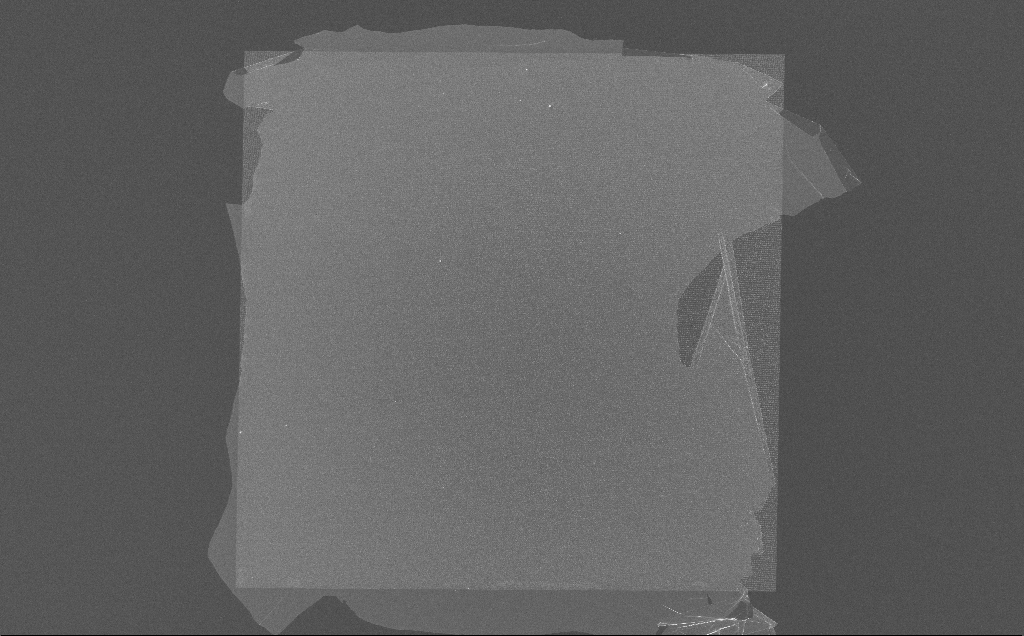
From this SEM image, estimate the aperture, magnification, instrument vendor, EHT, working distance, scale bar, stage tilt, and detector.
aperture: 30 µm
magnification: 0.268 K X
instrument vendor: Zeiss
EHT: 10 kV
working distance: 7 mm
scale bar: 100000 nm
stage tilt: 0°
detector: InLens